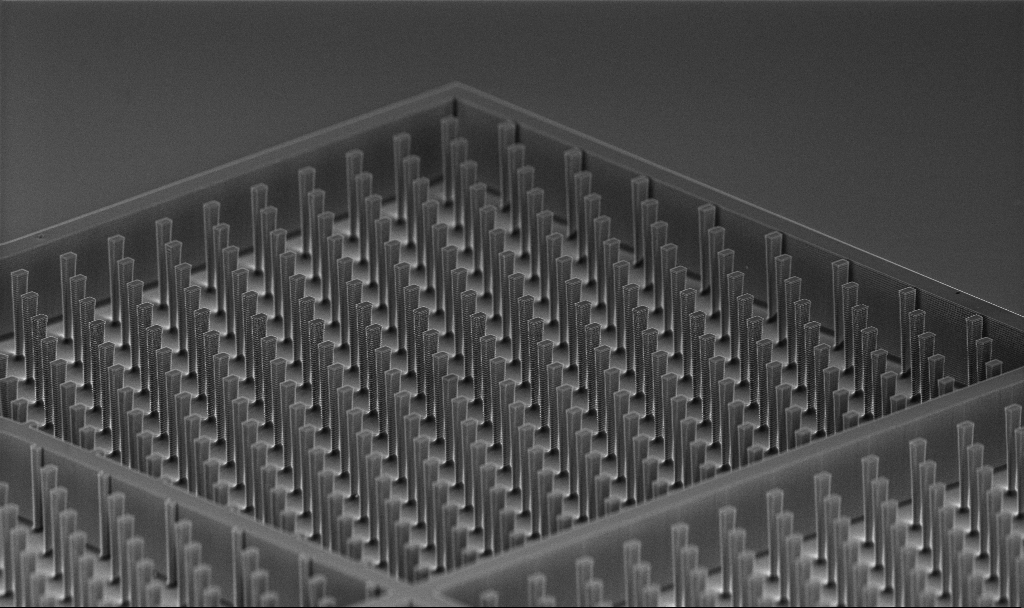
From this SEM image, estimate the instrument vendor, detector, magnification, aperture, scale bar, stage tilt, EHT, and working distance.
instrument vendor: Zeiss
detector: InLens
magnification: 2 K X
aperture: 30 µm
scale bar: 10000 nm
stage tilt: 70°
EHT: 5 kV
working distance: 6.2 mm